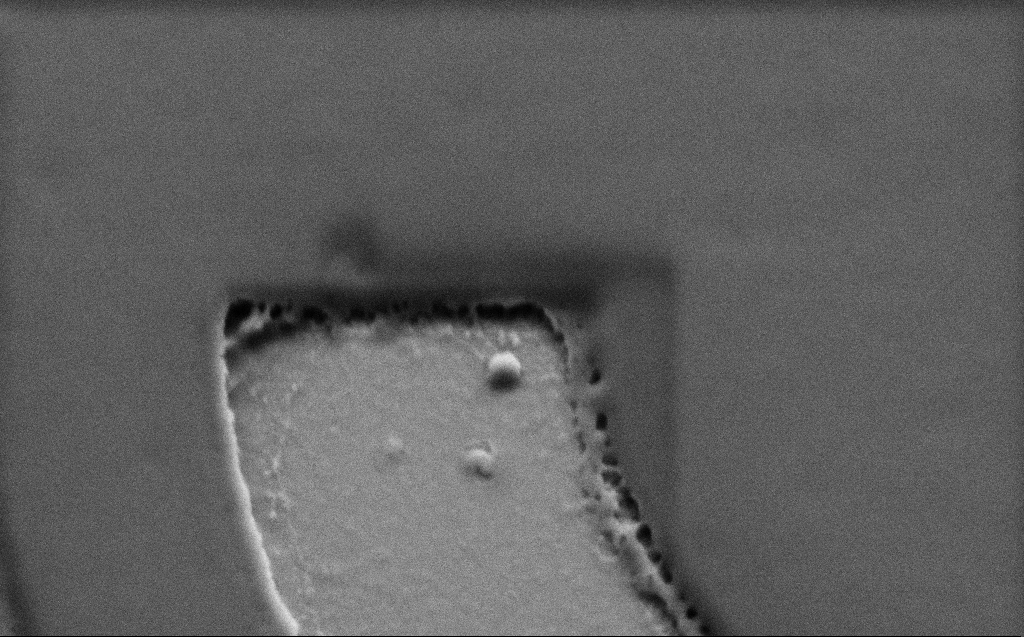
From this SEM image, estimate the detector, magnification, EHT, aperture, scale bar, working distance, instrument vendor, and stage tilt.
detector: SE2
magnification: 35.16 K X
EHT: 3 kV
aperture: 30 µm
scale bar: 2000 nm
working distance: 6 mm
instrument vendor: Zeiss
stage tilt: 45°